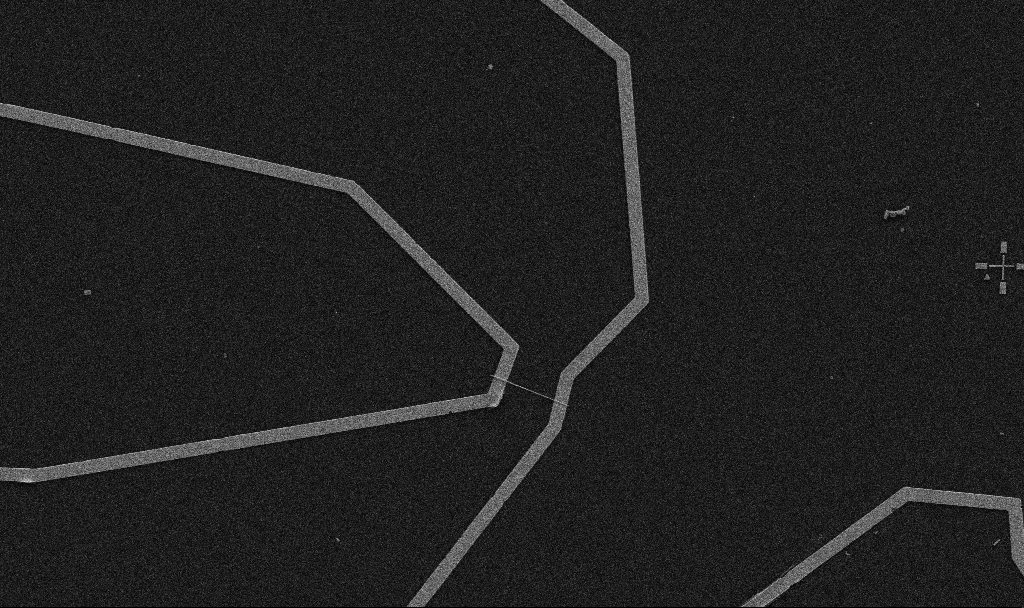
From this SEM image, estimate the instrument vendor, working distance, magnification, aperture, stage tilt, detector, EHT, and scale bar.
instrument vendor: Zeiss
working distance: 10.7 mm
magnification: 5 K X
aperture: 30 µm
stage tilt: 0°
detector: SE2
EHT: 5 kV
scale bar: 10000 nm